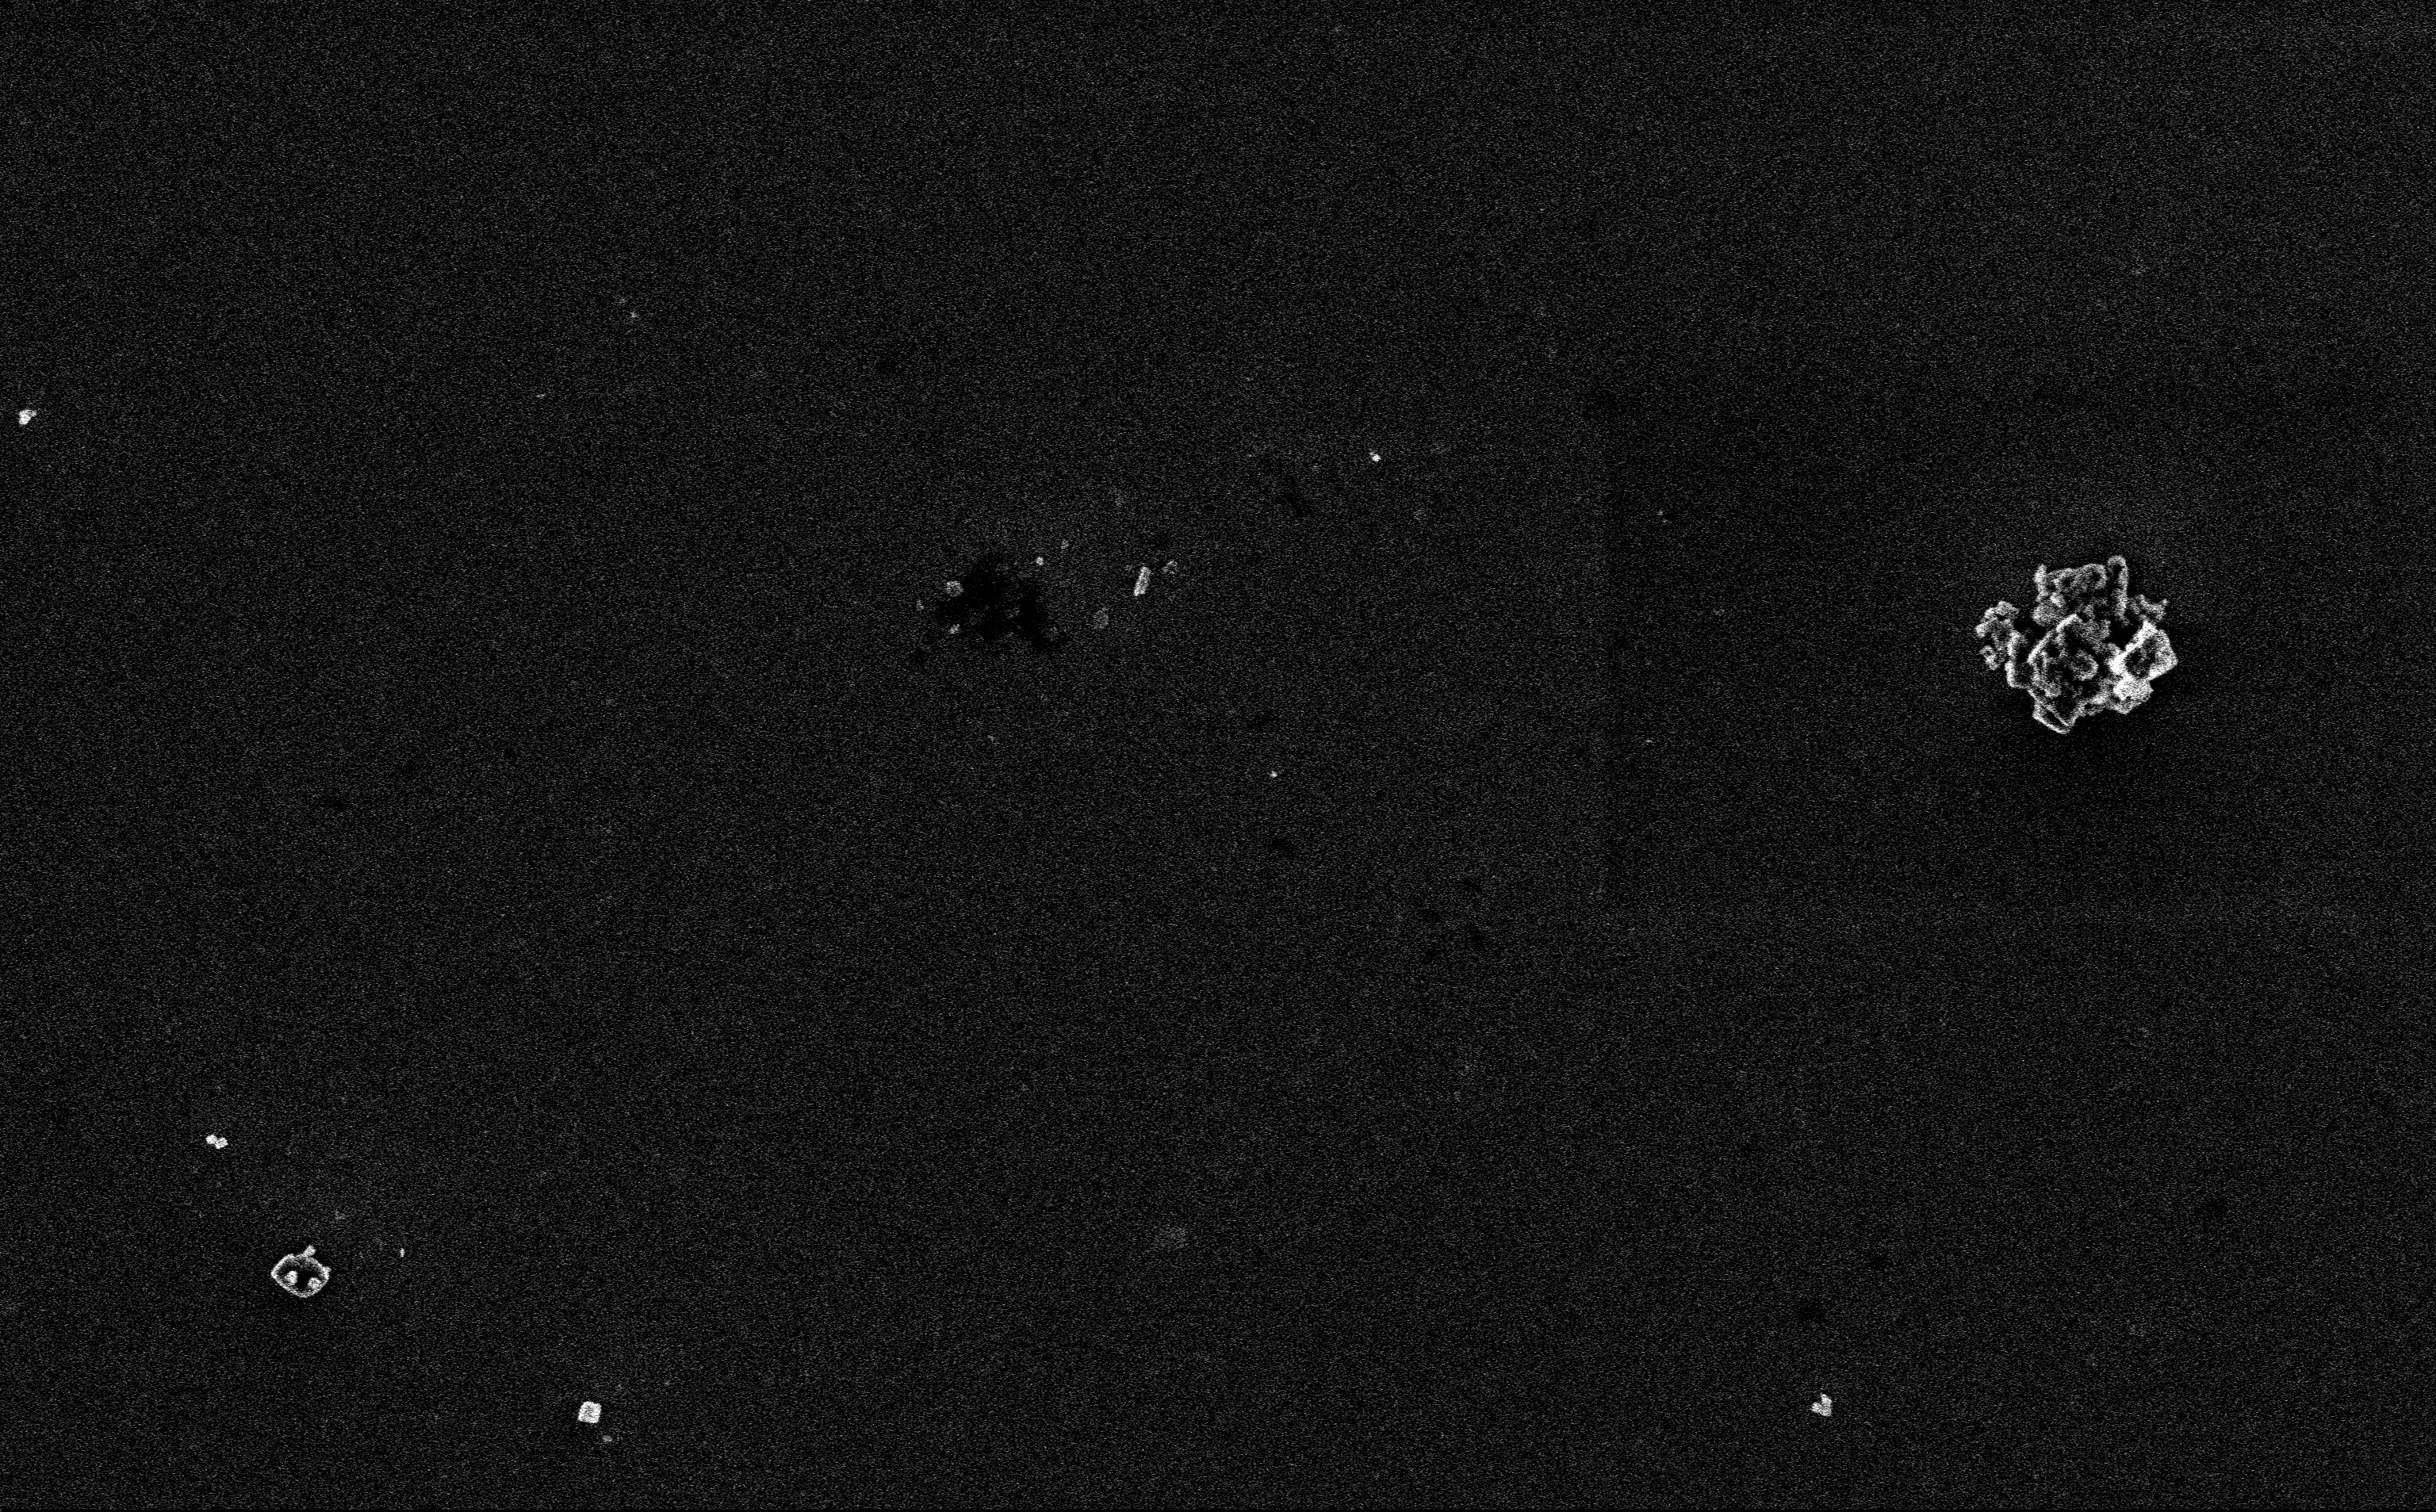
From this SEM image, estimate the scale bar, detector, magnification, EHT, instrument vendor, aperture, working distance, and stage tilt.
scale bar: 2000 nm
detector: InLens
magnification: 12.85 K X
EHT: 3 kV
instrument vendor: Zeiss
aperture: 30 µm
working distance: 3 mm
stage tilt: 0°